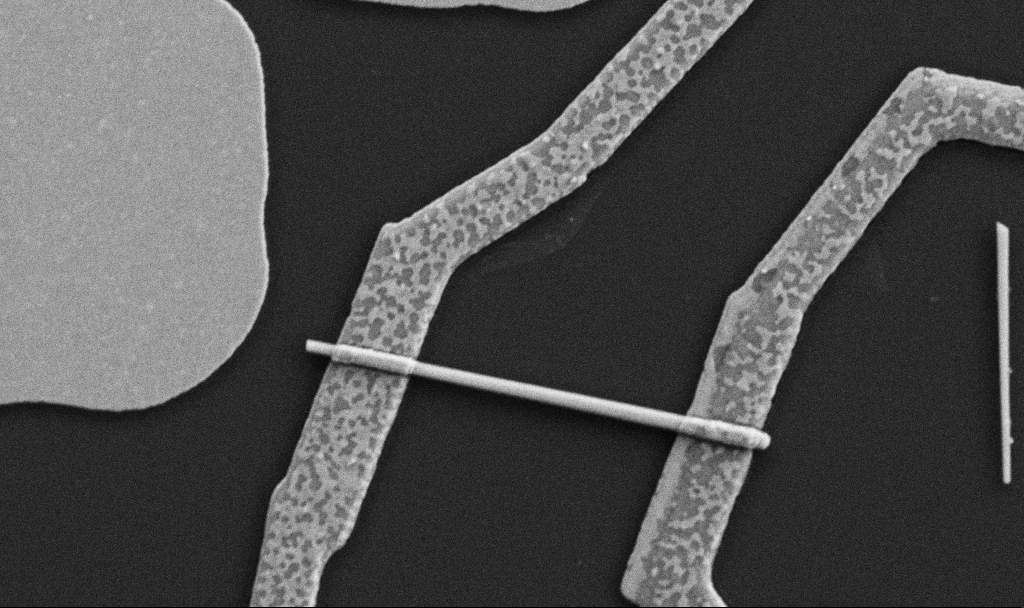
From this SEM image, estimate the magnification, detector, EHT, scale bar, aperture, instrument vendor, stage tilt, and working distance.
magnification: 30 K X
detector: SE2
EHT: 5 kV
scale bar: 1000 nm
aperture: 30 µm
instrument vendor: Zeiss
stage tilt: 0°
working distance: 8.7 mm